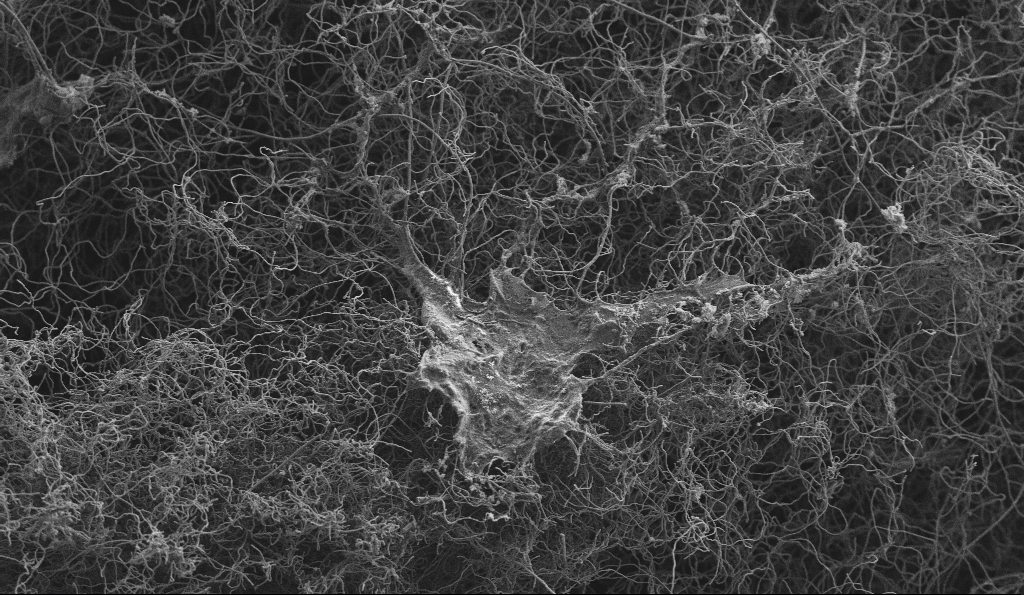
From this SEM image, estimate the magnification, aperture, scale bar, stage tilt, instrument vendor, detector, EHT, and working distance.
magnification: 2.5 K X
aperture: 30 µm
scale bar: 10000 nm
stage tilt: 0°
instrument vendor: Zeiss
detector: SE2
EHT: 3 kV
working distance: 5.2 mm